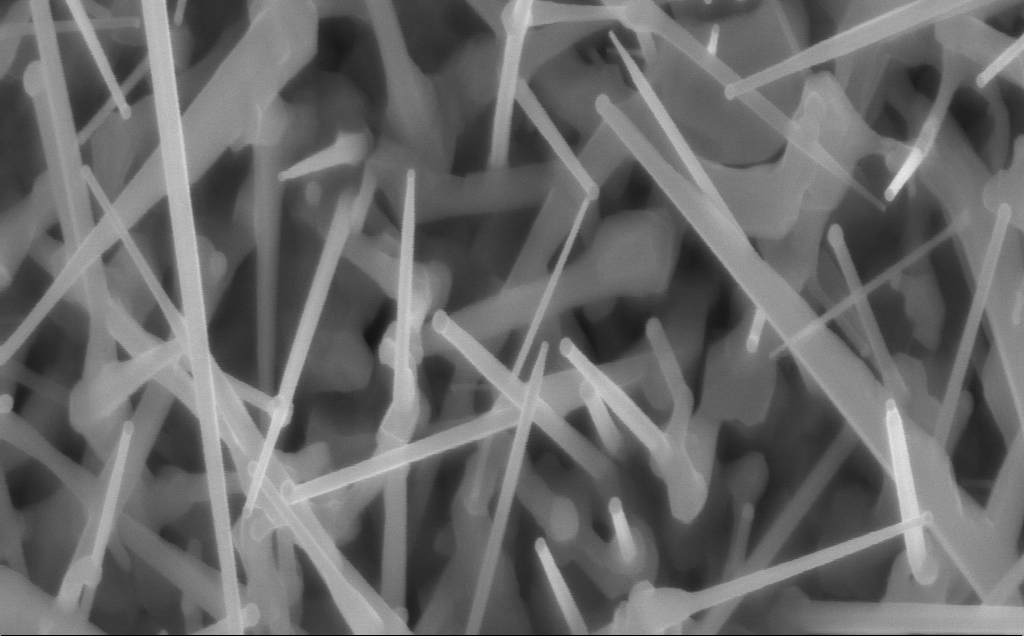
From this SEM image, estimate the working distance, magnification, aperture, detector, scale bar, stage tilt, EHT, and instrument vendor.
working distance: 4 mm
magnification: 80 K X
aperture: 30 µm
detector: InLens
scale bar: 200 nm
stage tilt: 0°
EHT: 10 kV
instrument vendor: Zeiss